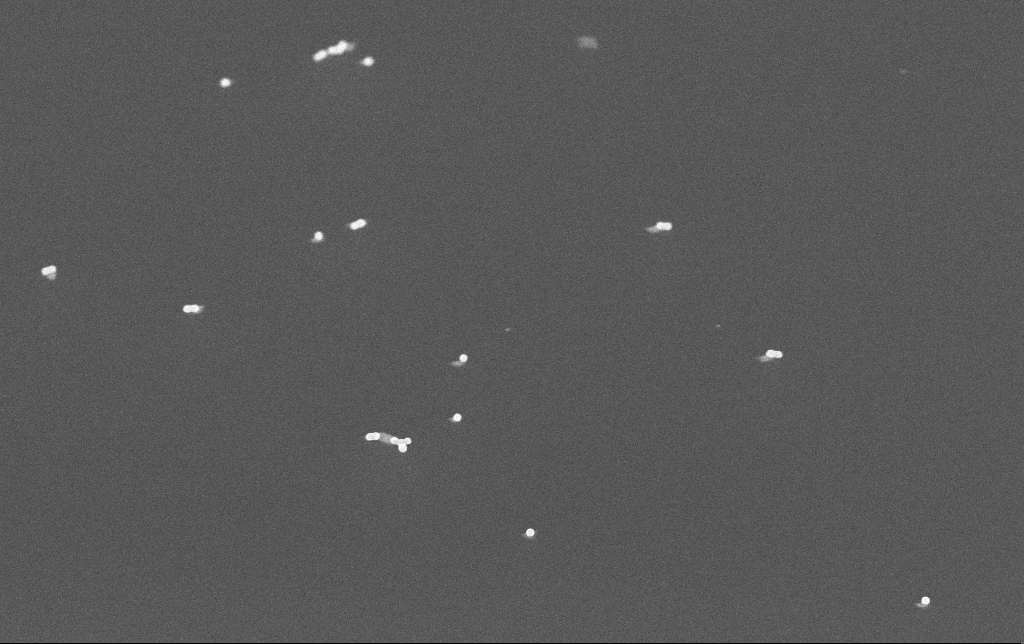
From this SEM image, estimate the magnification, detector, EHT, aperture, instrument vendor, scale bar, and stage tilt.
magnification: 50 K X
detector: InLens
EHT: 10 kV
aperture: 30 µm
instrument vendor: Zeiss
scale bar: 1000 nm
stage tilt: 45°